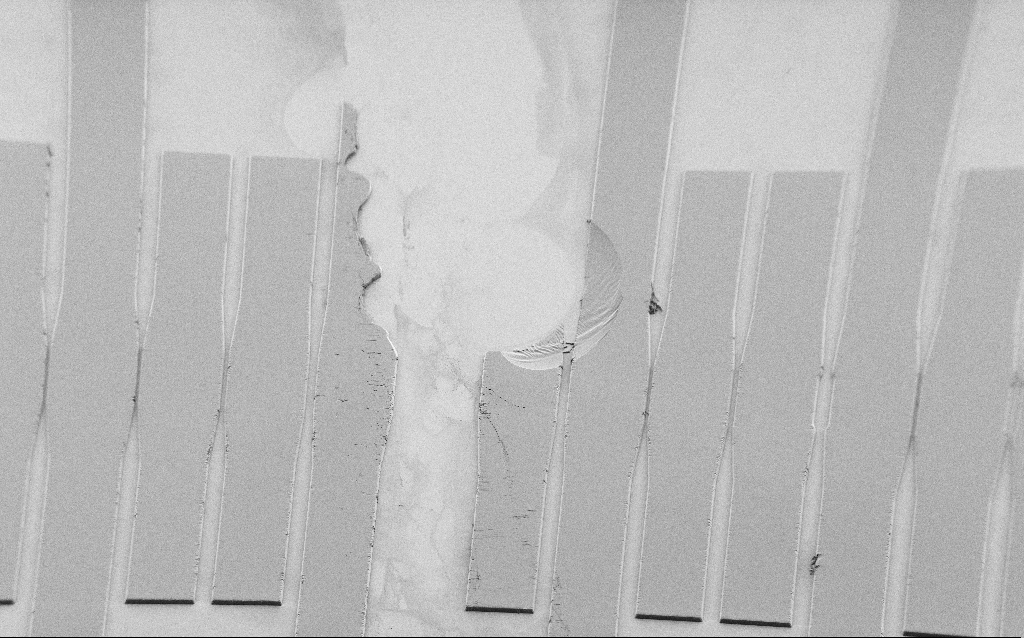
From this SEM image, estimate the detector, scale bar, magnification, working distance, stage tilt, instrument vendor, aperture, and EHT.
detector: SE2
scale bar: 100000 nm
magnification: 0.289 K X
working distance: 8 mm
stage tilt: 45°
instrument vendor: Zeiss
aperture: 30 µm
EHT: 1.2 kV